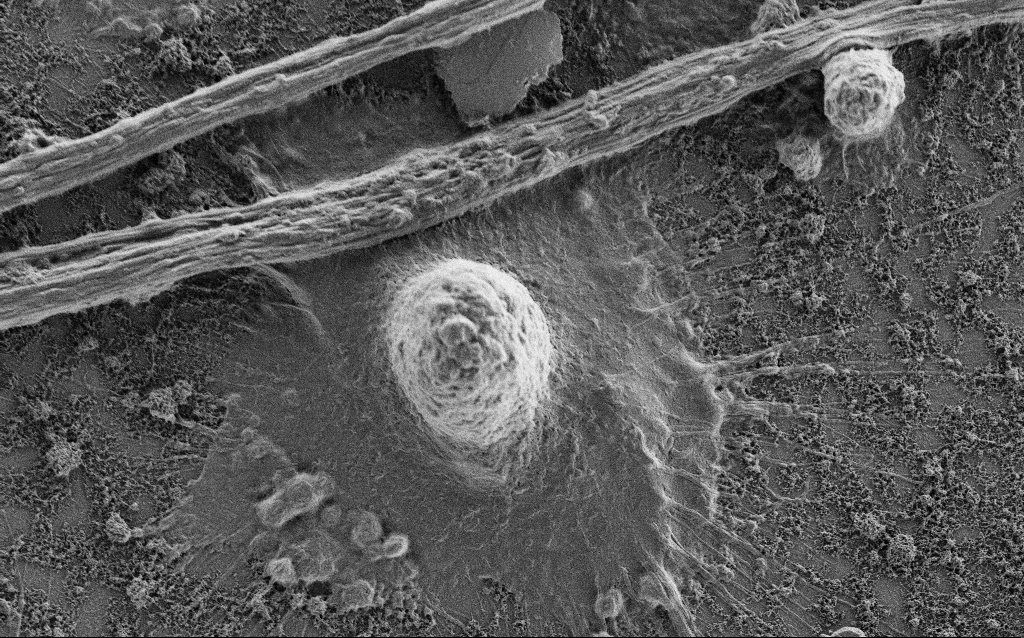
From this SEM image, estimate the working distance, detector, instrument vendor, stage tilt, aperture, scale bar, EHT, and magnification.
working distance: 4 mm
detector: SE2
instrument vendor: Zeiss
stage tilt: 0°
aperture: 30 µm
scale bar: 10000 nm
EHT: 0.9 kV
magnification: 5 K X